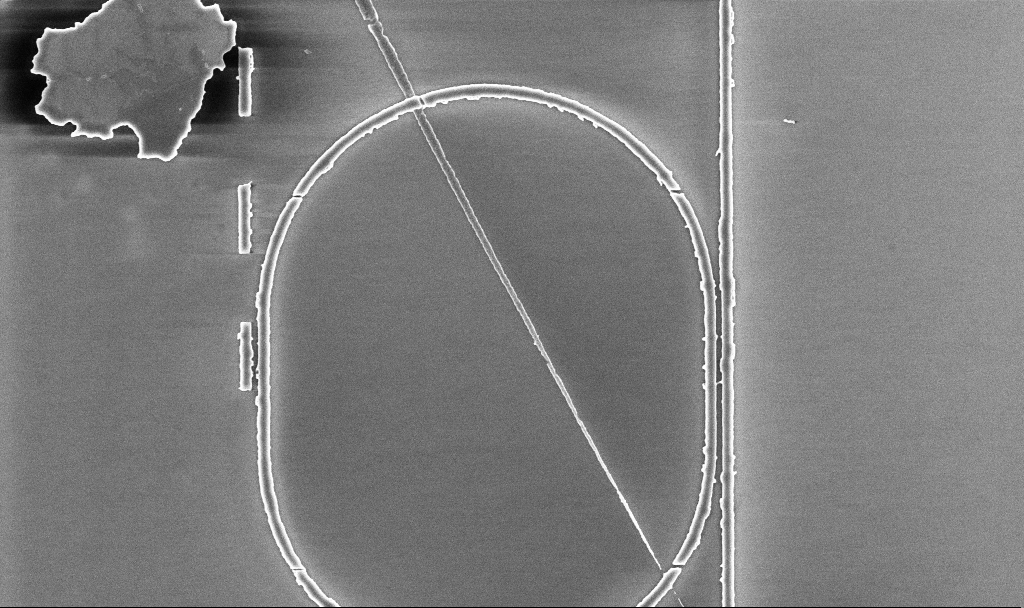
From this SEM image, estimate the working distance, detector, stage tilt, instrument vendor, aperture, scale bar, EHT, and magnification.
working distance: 5.2 mm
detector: InLens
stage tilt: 0°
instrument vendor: Zeiss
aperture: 30 µm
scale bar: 2000 nm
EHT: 5 kV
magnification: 8.51 K X